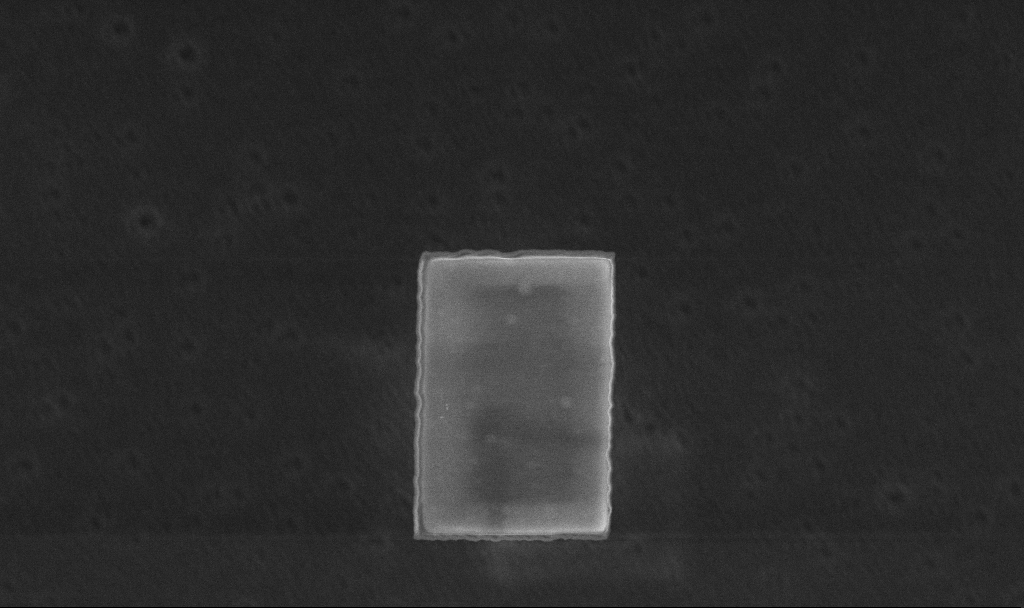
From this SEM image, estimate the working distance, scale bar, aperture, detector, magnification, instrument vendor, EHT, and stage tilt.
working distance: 4.8 mm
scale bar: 1000 nm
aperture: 30 µm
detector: InLens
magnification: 24.56 K X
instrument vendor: Zeiss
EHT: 5 kV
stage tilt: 0°